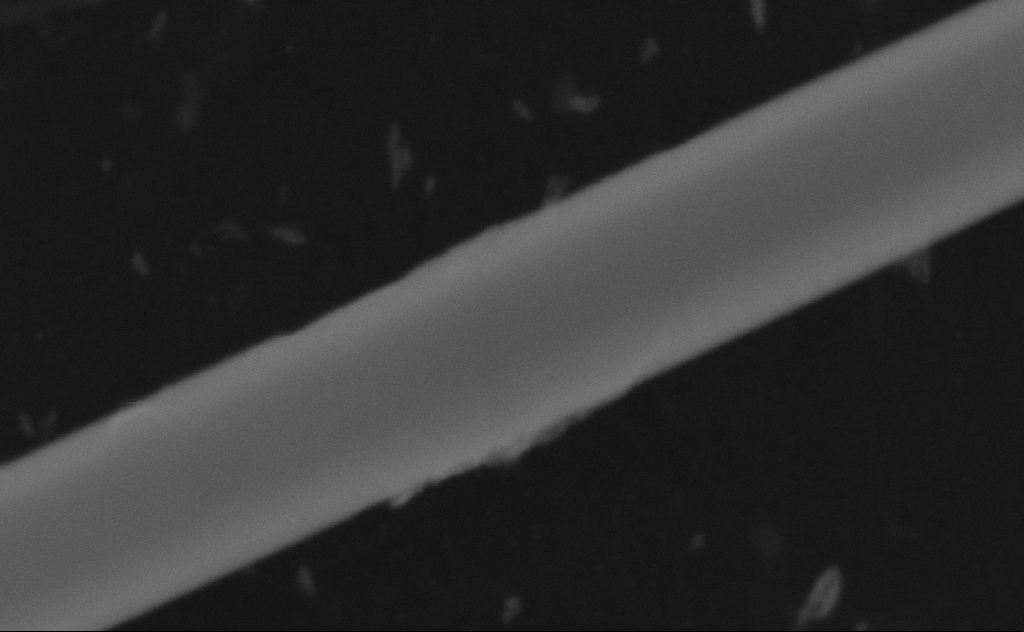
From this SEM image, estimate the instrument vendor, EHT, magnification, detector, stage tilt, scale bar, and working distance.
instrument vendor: Zeiss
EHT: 20 kV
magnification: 454.62 K X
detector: SE2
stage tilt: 0°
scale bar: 100 nm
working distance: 8 mm